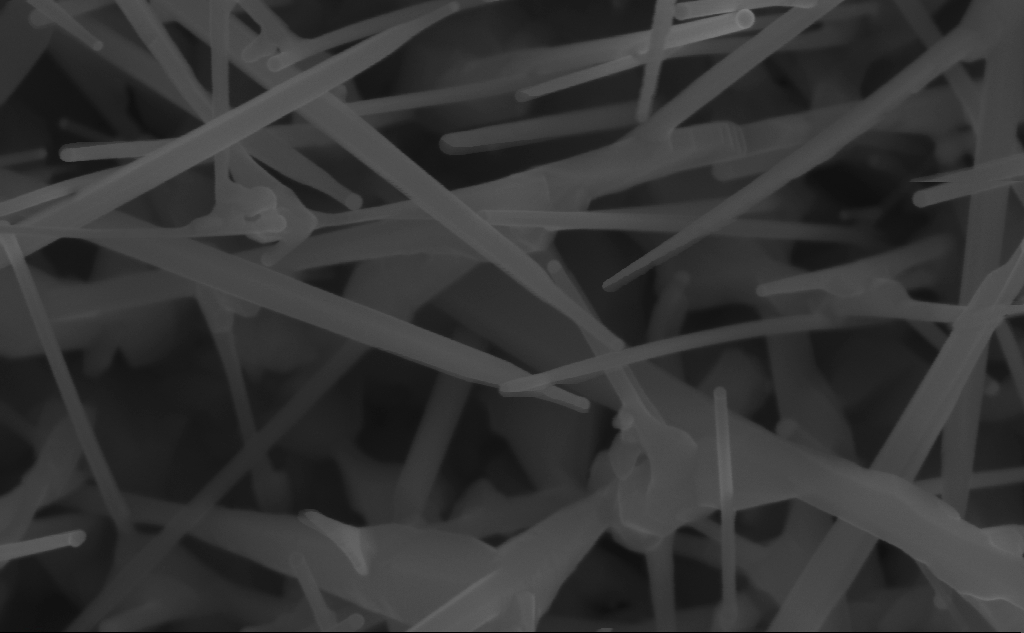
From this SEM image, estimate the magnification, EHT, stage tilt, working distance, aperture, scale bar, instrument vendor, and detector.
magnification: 168.76 K X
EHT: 10 kV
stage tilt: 0°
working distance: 7 mm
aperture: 30 µm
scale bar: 100 nm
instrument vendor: Zeiss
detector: InLens